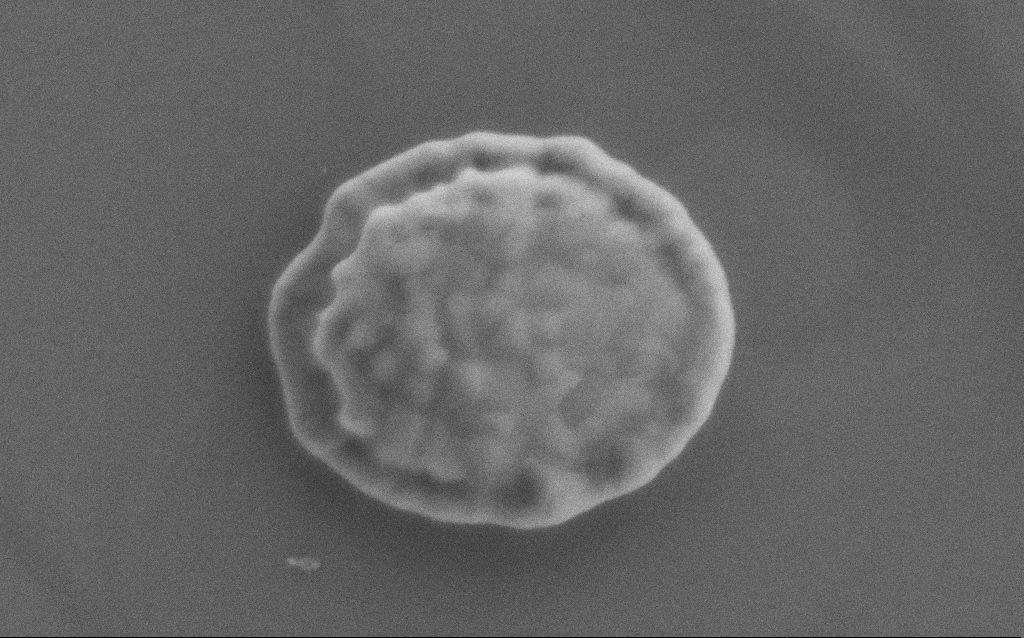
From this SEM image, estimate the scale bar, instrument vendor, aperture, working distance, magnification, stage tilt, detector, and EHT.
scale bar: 200 nm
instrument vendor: Zeiss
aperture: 30 µm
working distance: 2 mm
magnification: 88.38 K X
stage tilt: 0°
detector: SE2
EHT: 5 kV